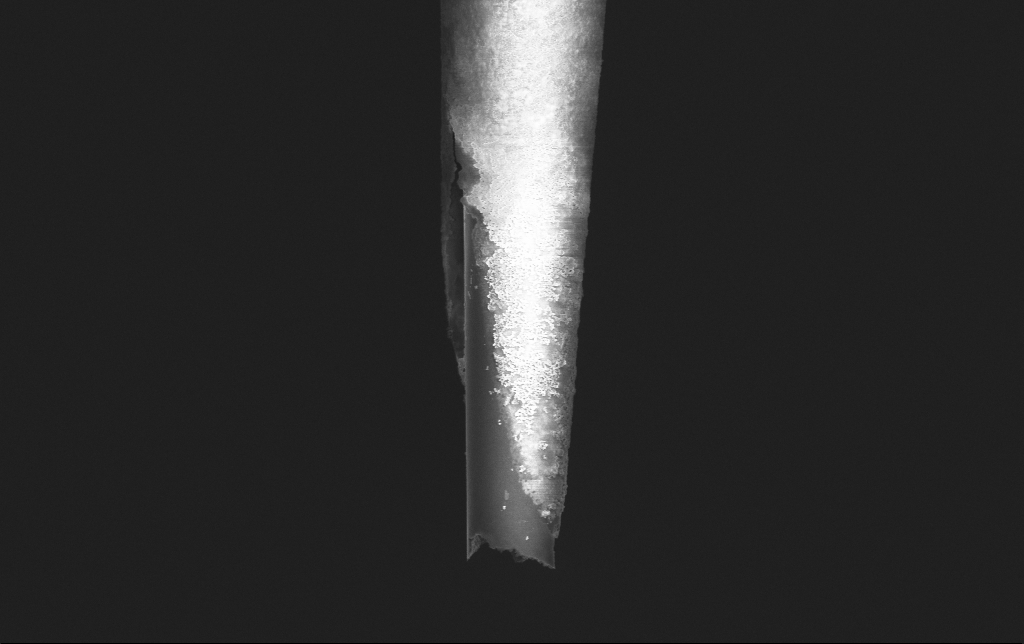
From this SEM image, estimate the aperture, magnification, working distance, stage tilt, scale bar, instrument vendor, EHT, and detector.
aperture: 30 µm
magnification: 25 K X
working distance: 5.9 mm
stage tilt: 0°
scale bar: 2000 nm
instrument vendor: Zeiss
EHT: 2 kV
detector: InLens